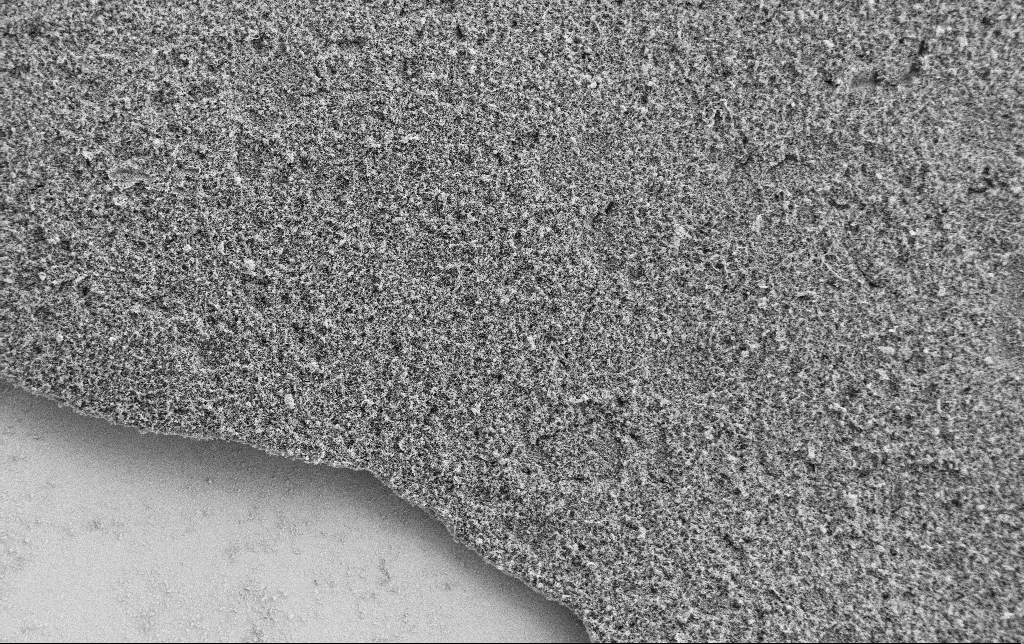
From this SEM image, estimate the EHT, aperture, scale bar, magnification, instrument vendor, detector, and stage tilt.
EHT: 2 kV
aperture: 30 µm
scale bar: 100000 nm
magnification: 0.5 K X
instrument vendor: Zeiss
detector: SE2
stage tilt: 0°